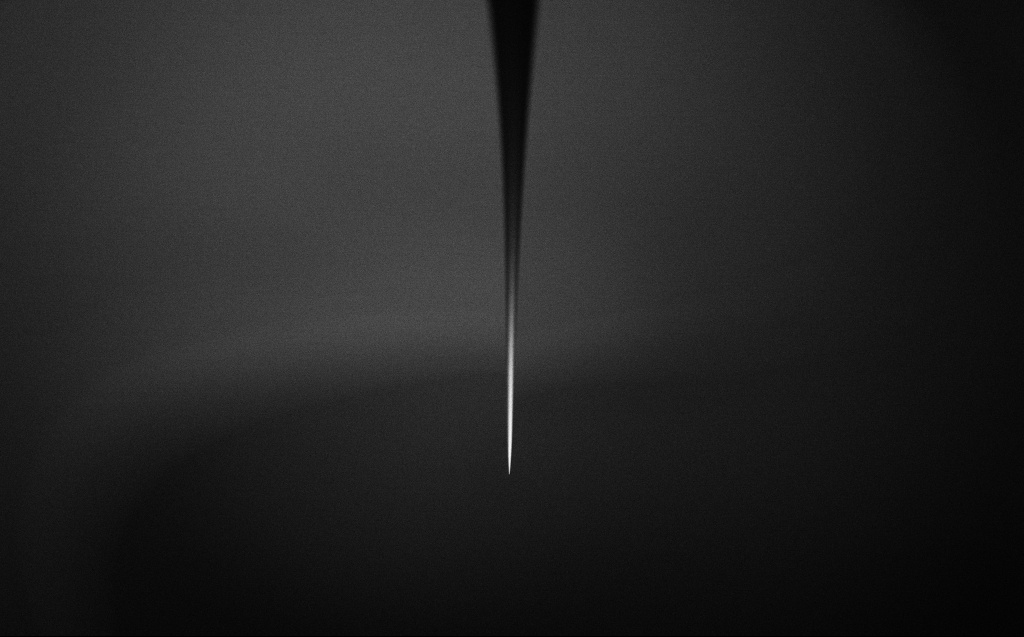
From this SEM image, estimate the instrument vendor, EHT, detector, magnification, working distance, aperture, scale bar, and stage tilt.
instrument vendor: Zeiss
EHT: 2 kV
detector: InLens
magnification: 0.1 K X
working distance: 4 mm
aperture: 30 µm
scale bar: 200000 nm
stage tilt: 45°